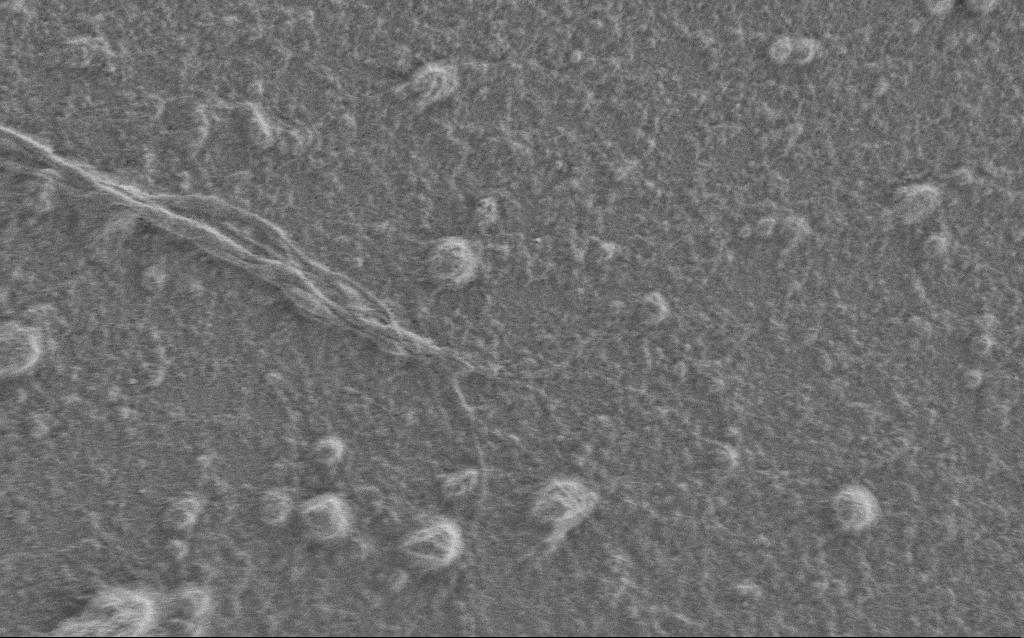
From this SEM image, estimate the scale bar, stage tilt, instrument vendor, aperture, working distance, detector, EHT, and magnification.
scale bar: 2000 nm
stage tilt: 0°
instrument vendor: Zeiss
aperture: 30 µm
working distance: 6 mm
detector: SE2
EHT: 1 kV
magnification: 7.5 K X